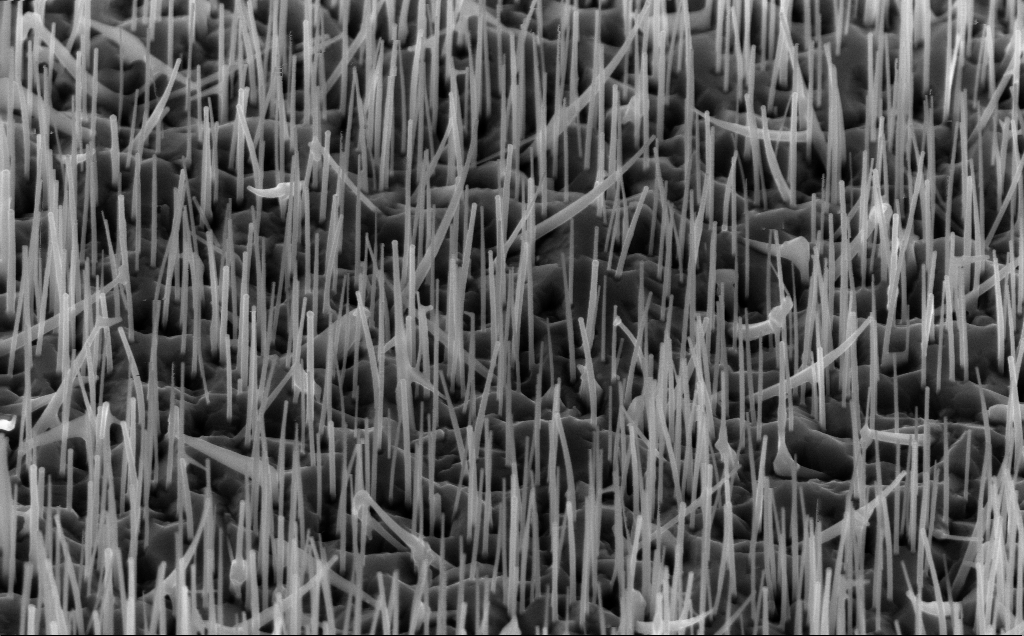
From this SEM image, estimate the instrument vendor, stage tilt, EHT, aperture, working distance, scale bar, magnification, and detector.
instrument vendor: Zeiss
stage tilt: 45°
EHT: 10 kV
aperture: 30 µm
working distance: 5 mm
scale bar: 1000 nm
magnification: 40 K X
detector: InLens